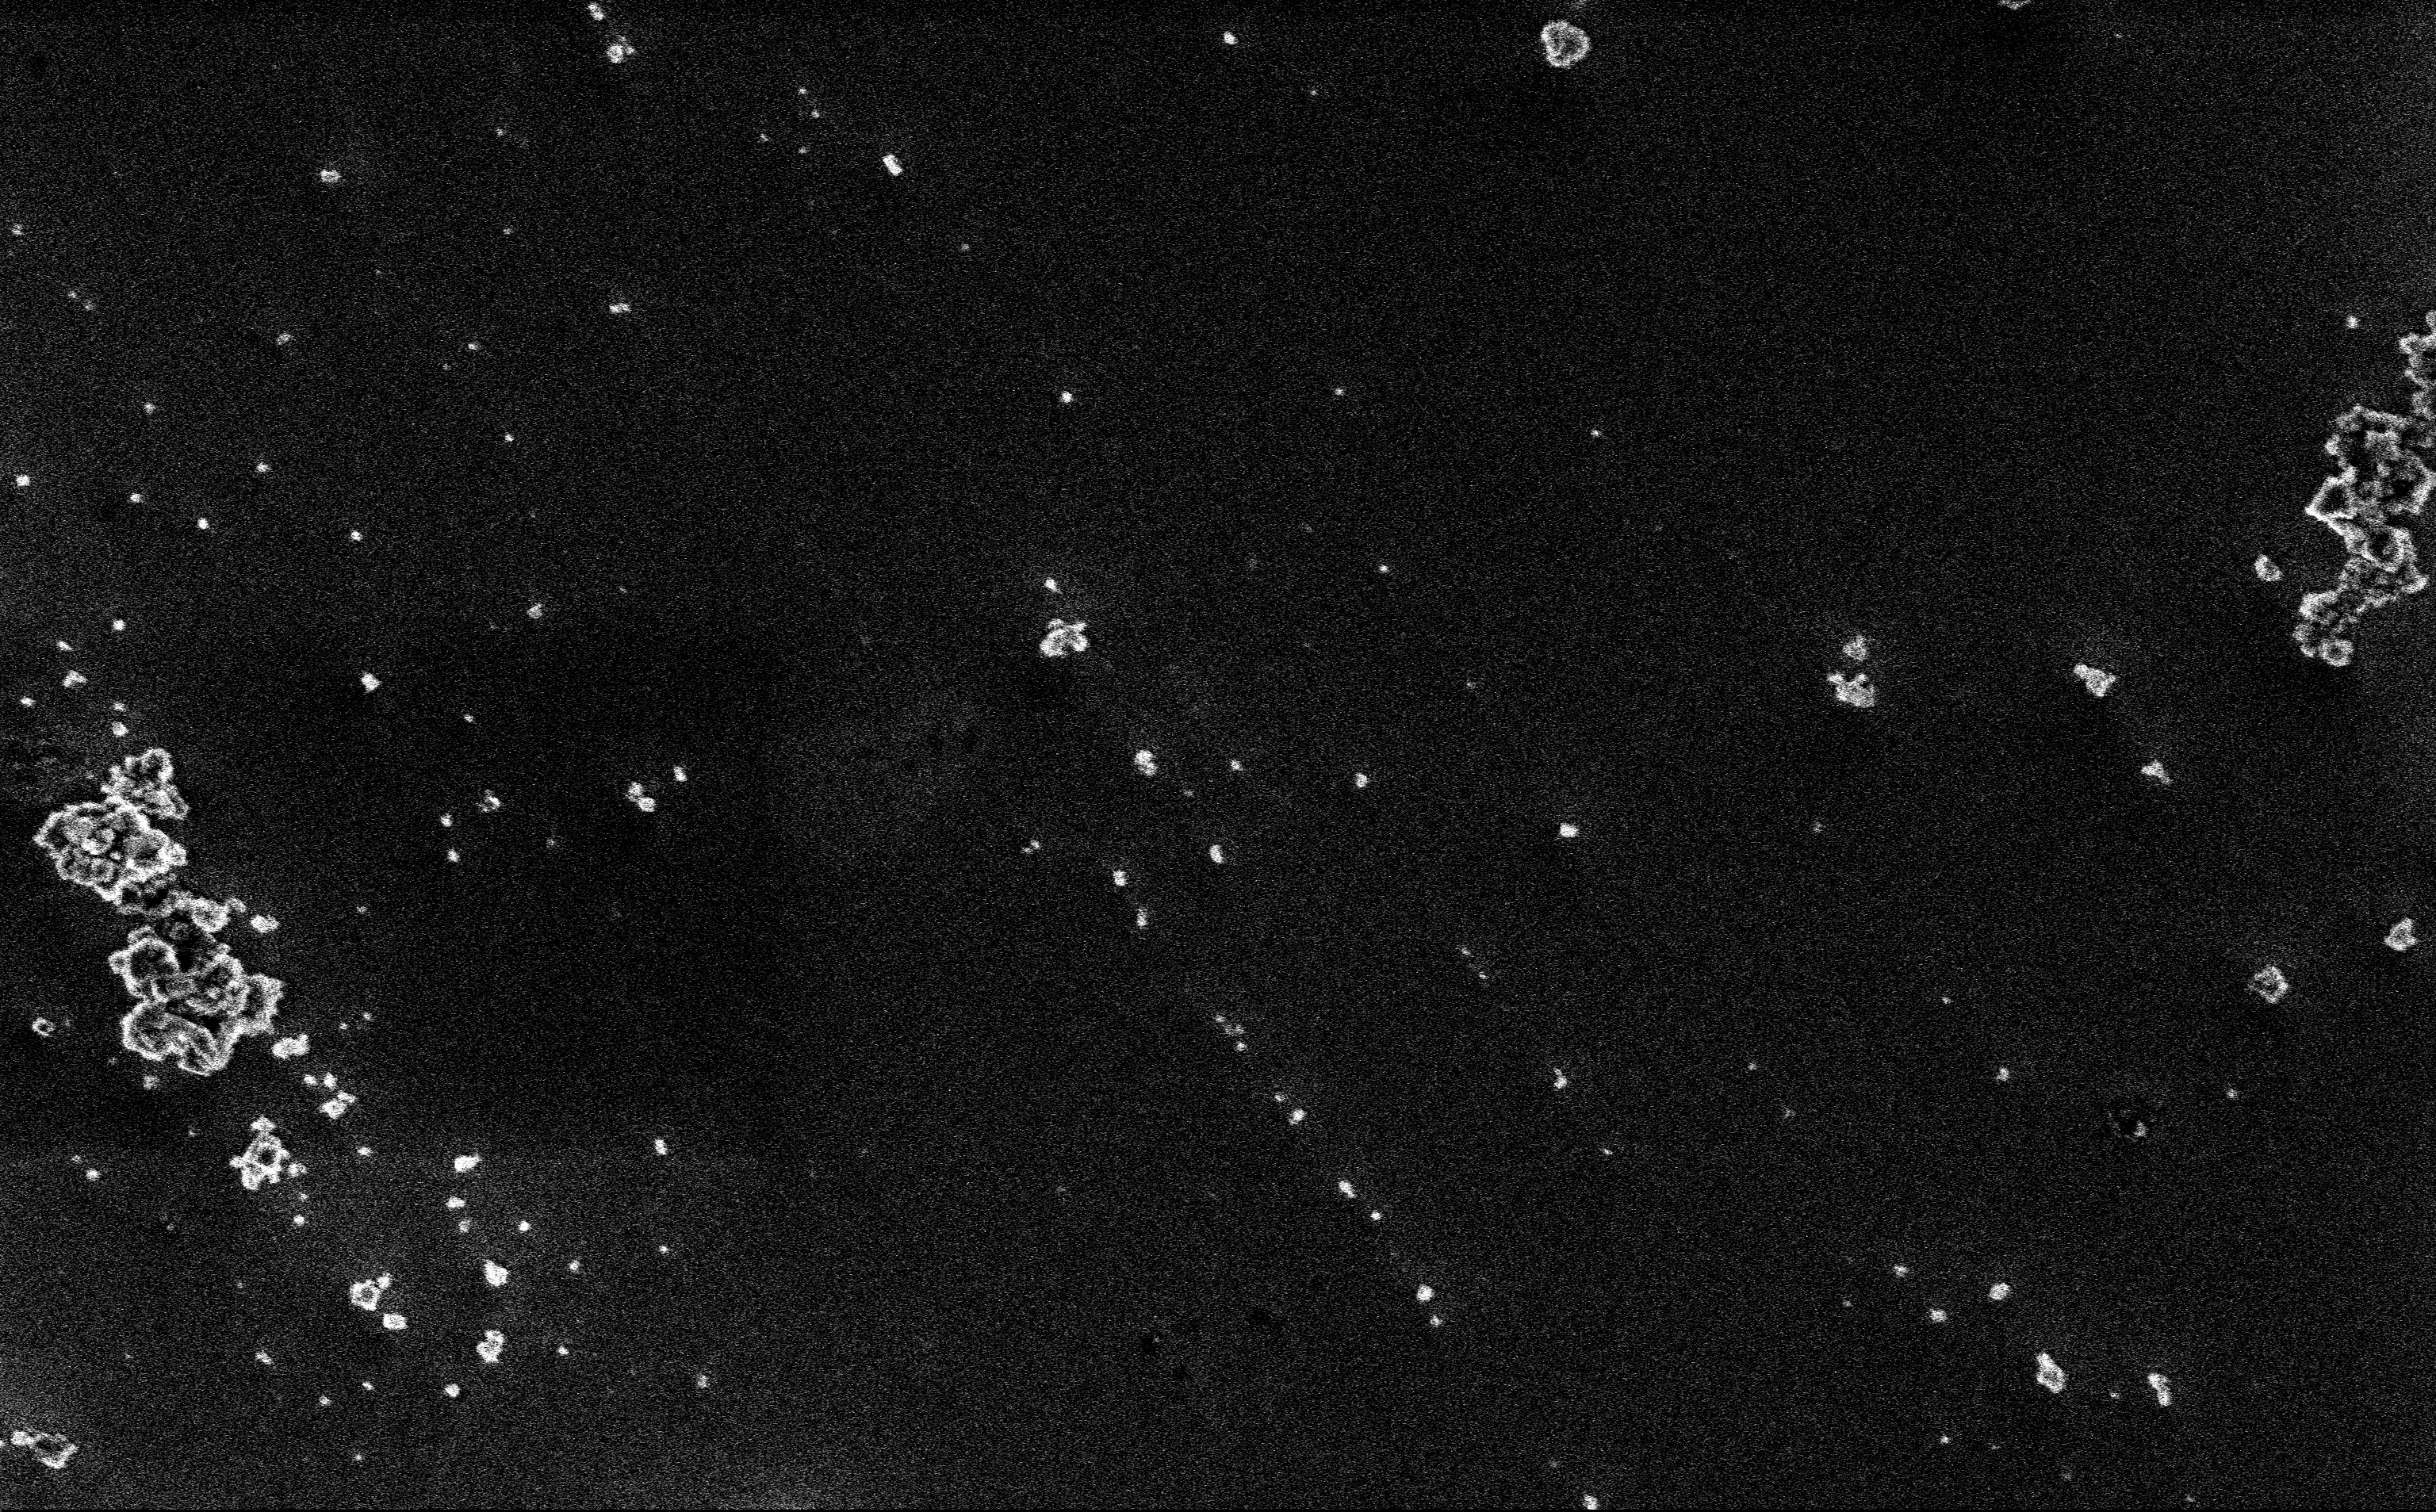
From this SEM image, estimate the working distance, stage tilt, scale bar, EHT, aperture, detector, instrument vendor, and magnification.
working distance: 3 mm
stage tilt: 0°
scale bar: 2000 nm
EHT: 3 kV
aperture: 30 µm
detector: InLens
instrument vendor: Zeiss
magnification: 12.85 K X